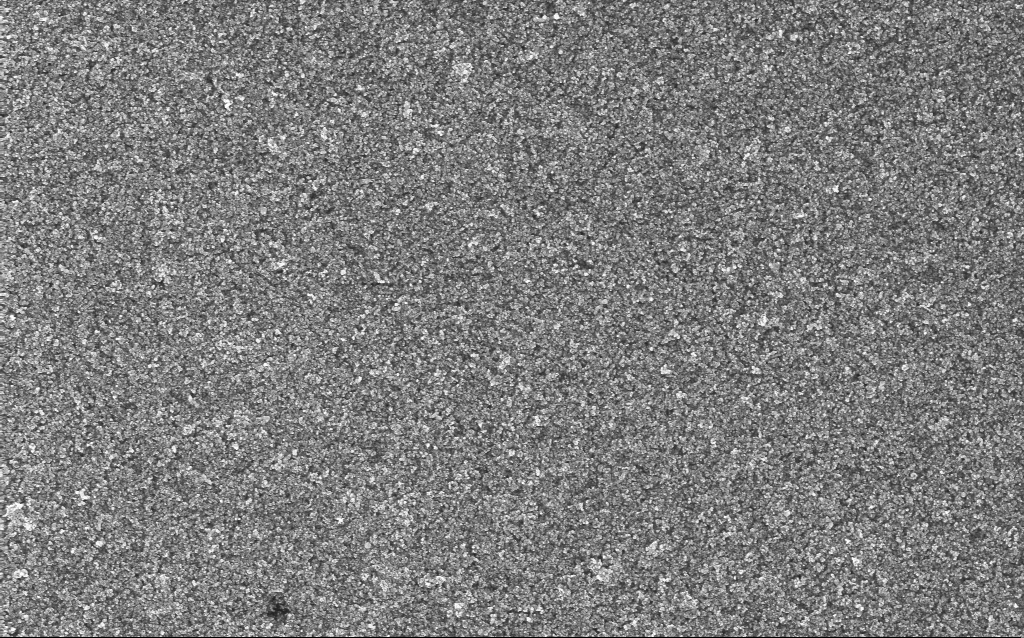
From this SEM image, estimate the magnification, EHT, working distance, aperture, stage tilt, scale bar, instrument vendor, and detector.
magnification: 20.87 K X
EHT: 5 kV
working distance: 6.4 mm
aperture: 30 µm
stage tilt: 0°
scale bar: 1000 nm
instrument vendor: Zeiss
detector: InLens